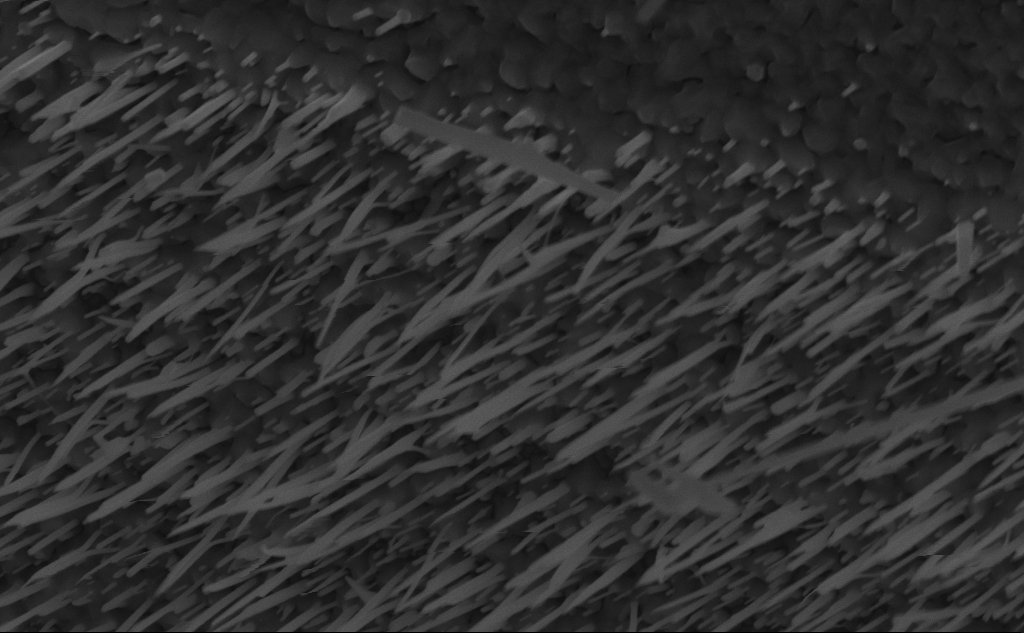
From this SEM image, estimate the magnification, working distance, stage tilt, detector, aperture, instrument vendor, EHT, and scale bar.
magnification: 57.23 K X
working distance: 5 mm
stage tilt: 45°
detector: InLens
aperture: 30 µm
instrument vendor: Zeiss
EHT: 10 kV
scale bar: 1000 nm